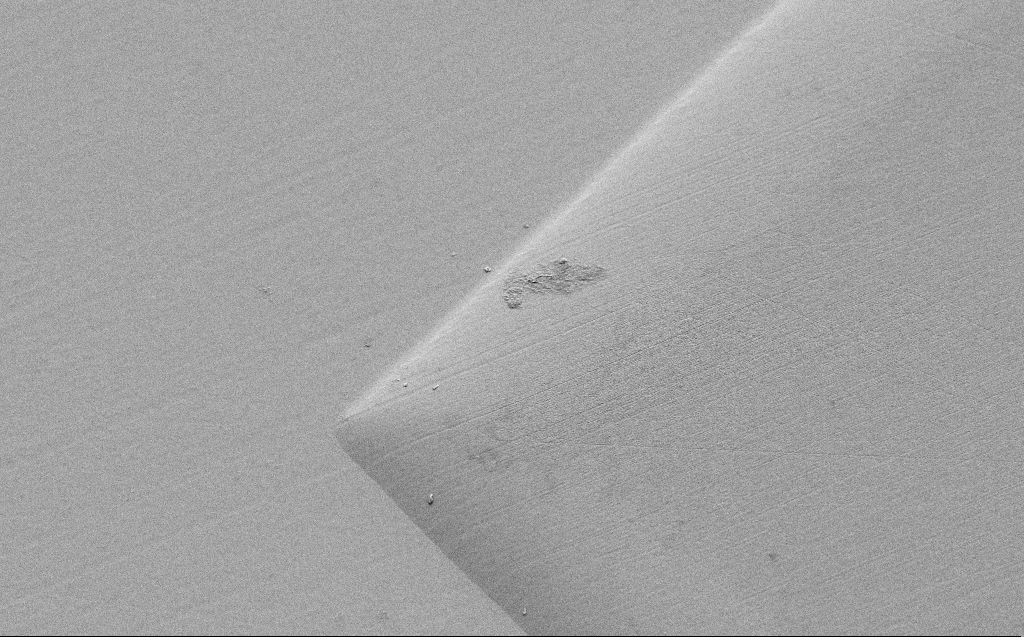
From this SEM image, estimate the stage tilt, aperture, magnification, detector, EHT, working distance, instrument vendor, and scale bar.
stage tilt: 45°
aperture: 30 µm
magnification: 1.5 K X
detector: SE2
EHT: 5 kV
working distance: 8 mm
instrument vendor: Zeiss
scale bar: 20000 nm